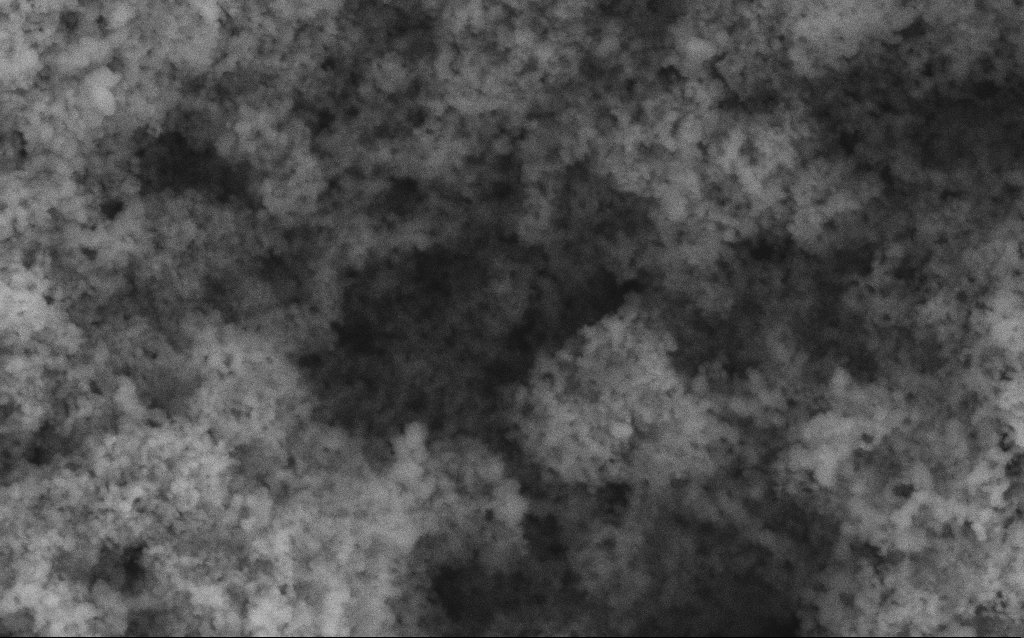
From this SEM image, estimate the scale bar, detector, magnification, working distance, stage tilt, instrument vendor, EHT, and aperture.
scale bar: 200 nm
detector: SE2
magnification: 114.64 K X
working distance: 4.2 mm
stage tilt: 0°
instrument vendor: Zeiss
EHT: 5 kV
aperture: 30 µm